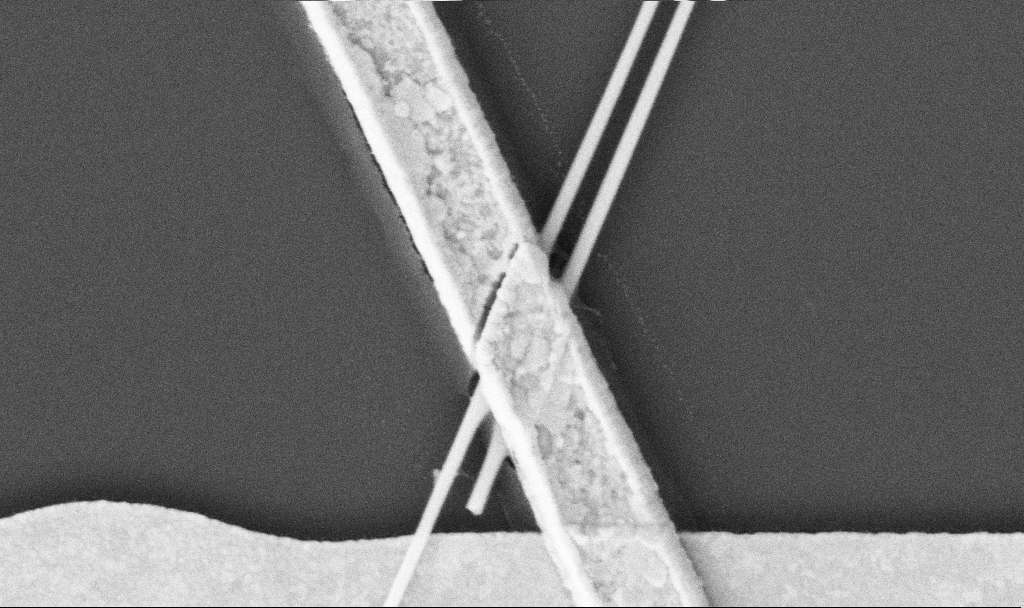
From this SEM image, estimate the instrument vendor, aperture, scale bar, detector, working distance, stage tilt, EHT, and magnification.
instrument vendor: Zeiss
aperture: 30 µm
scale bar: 1000 nm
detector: SE2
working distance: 10.7 mm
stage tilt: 0°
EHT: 5 kV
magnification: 60 K X